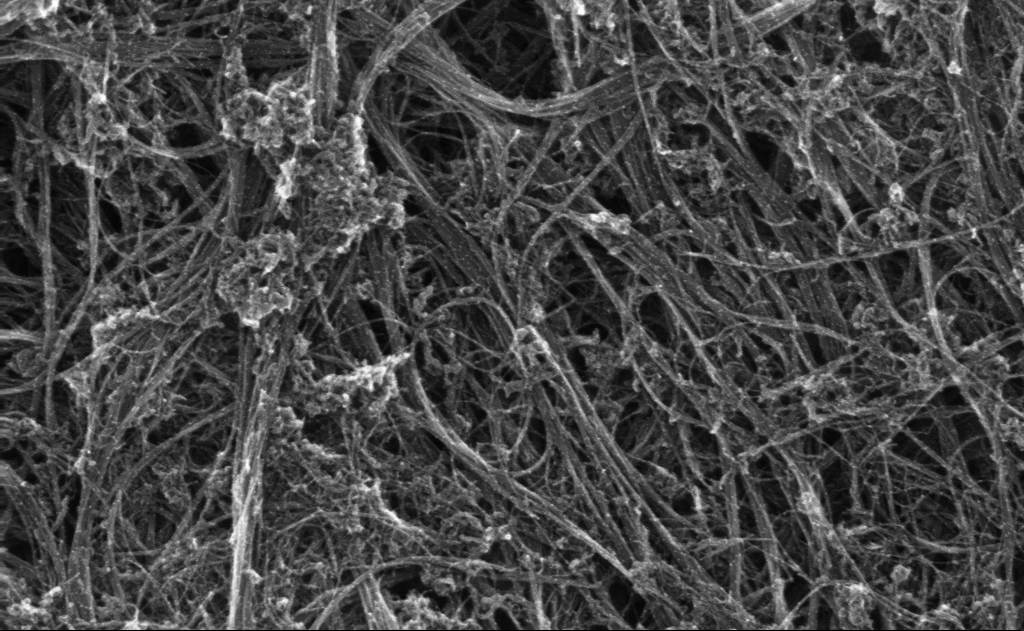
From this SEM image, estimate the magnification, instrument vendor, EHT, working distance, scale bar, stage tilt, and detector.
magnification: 173.01 K X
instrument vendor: Zeiss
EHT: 10 kV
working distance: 3 mm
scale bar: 100 nm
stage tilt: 0°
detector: InLens